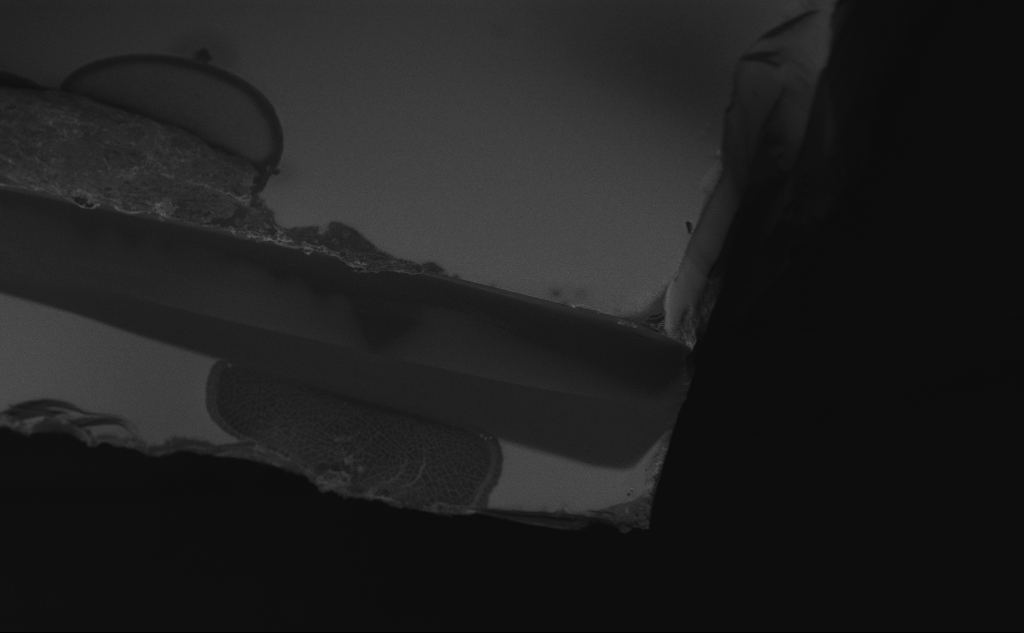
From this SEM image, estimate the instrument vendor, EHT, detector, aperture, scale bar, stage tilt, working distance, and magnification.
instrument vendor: Zeiss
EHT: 10 kV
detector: InLens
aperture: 30 µm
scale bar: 100000 nm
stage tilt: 45°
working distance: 5 mm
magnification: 0.247 K X